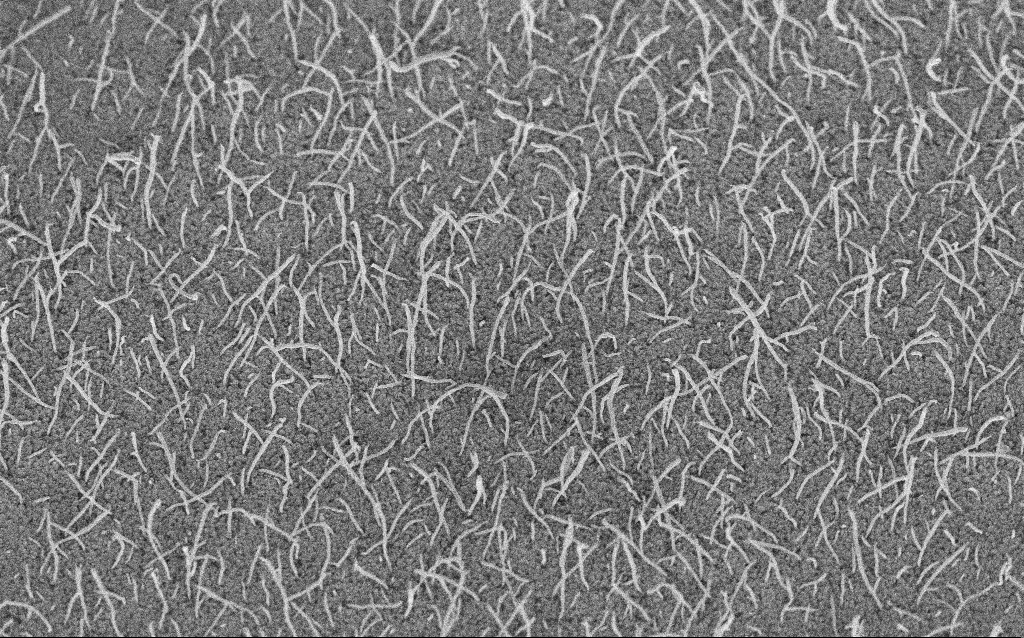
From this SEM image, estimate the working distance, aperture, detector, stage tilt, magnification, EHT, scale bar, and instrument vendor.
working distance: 8.7 mm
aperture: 30 µm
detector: InLens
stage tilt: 45°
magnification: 20 K X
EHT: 5 kV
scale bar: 1000 nm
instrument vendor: Zeiss